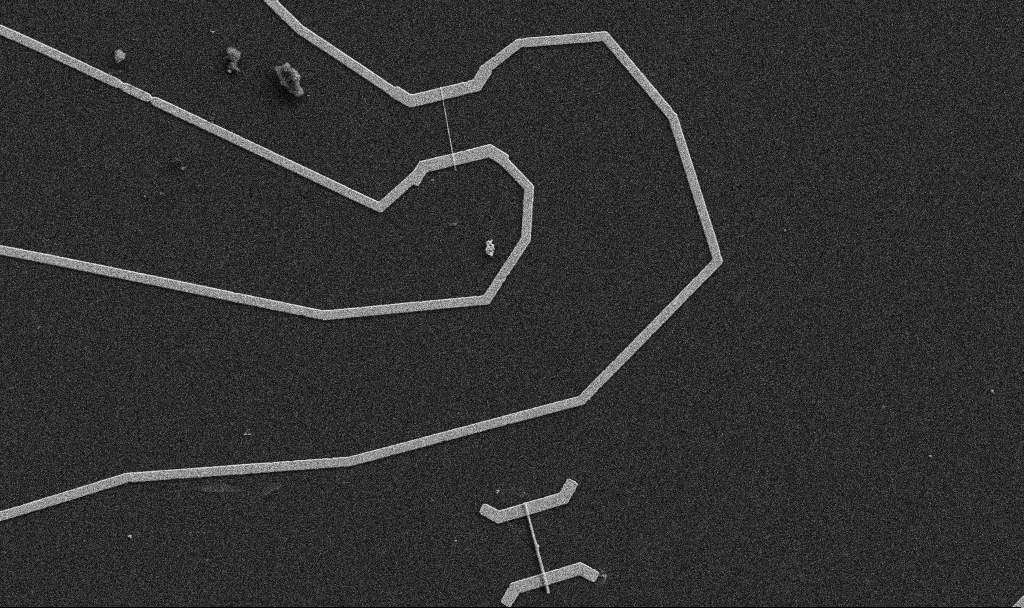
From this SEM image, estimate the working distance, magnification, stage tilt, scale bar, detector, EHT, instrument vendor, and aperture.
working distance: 10.7 mm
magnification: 5 K X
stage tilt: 0°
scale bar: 10000 nm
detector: SE2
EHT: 5 kV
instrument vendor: Zeiss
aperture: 30 µm